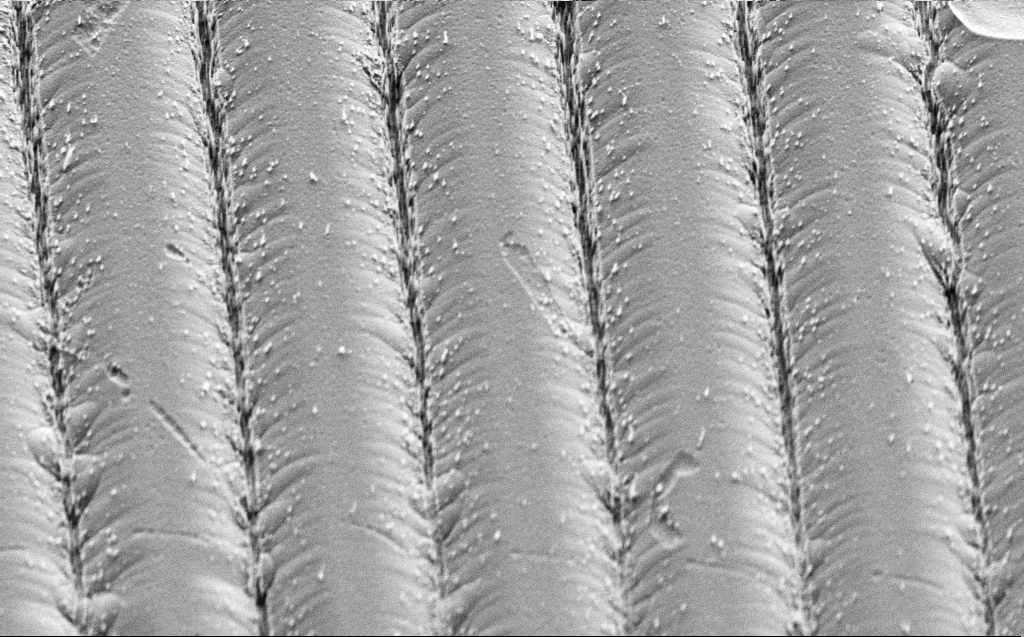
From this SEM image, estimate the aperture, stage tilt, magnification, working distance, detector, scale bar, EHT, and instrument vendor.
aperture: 30 µm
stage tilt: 45°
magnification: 6.61 K X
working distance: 9 mm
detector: SE2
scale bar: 10000 nm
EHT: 3 kV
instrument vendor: Zeiss